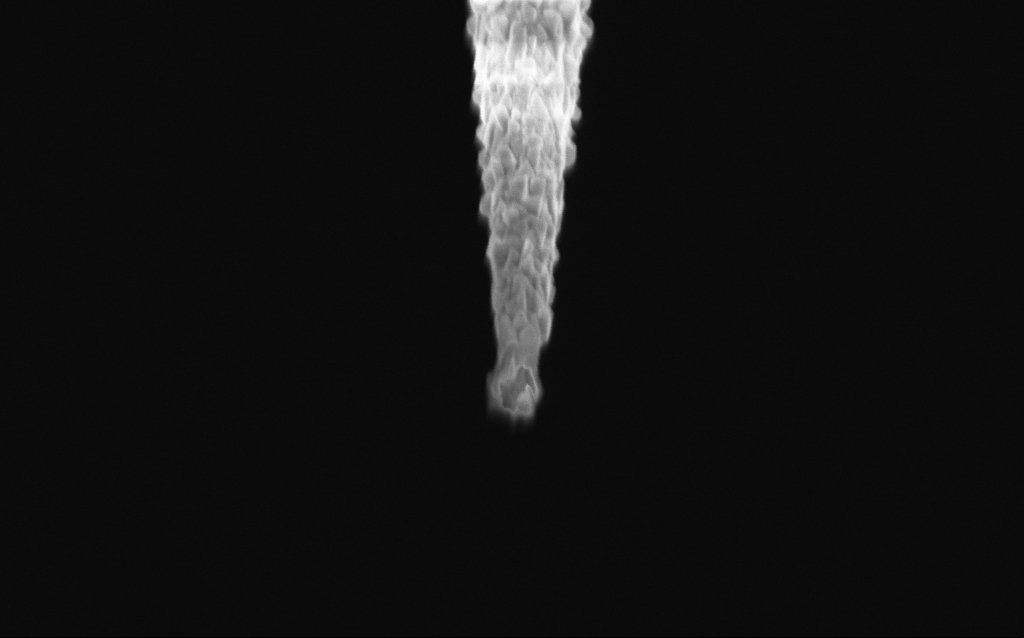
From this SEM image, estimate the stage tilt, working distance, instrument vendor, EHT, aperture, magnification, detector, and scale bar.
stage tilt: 44.9°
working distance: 6.2 mm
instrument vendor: Zeiss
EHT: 2 kV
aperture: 30 µm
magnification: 50 K X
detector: InLens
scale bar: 1000 nm